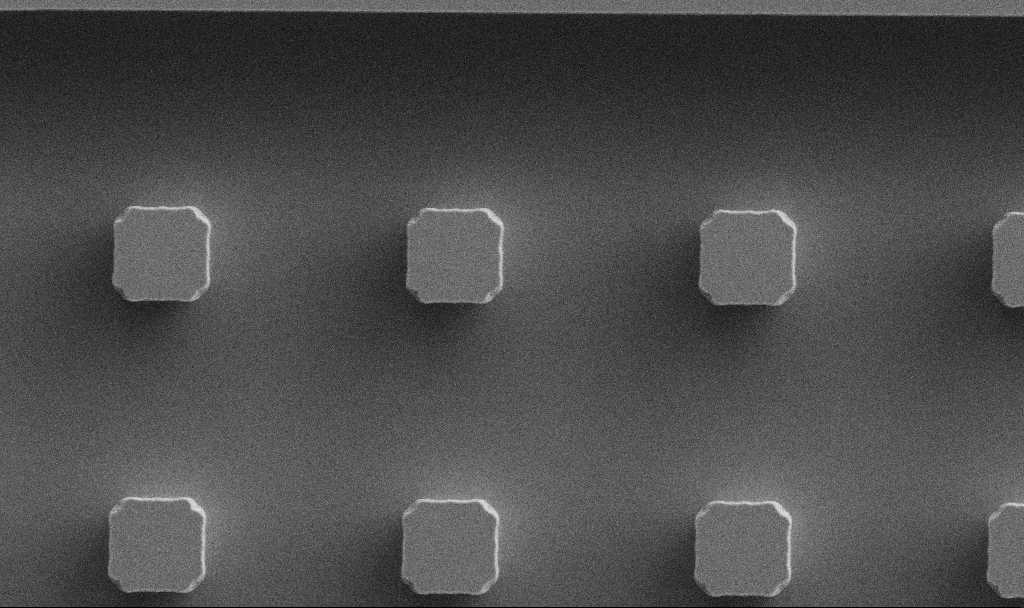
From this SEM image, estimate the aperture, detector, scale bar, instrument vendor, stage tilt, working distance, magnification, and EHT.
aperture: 30 µm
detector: SE2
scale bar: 10000 nm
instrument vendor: Zeiss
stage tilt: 0°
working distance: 5.3 mm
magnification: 3.6 K X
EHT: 5 kV